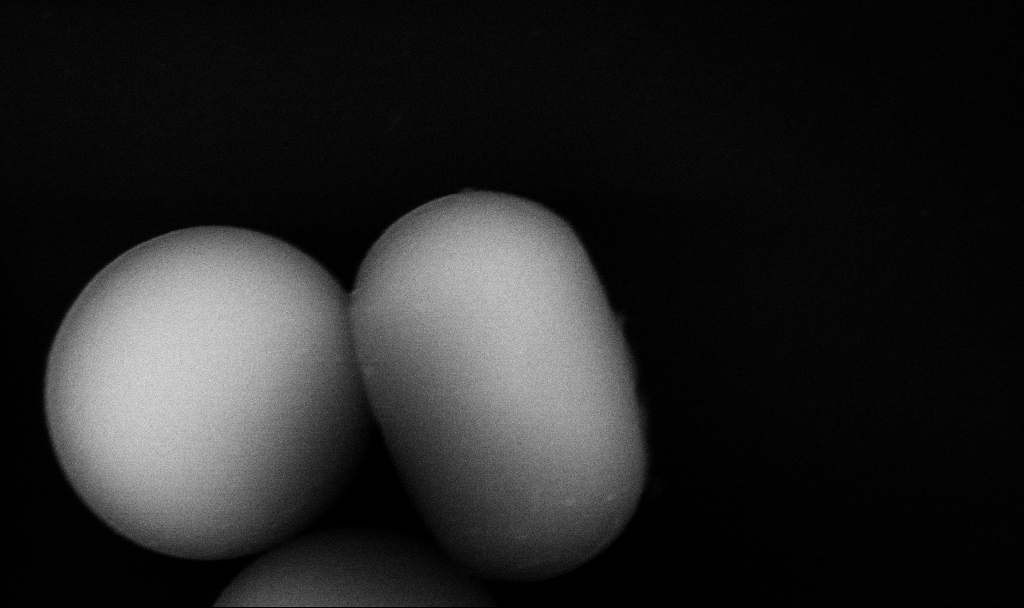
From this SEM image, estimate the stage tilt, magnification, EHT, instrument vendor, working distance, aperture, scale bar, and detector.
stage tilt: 0°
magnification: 59.13 K X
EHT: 10 kV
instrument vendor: Zeiss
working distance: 5.2 mm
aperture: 30 µm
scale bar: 1000 nm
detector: InLens